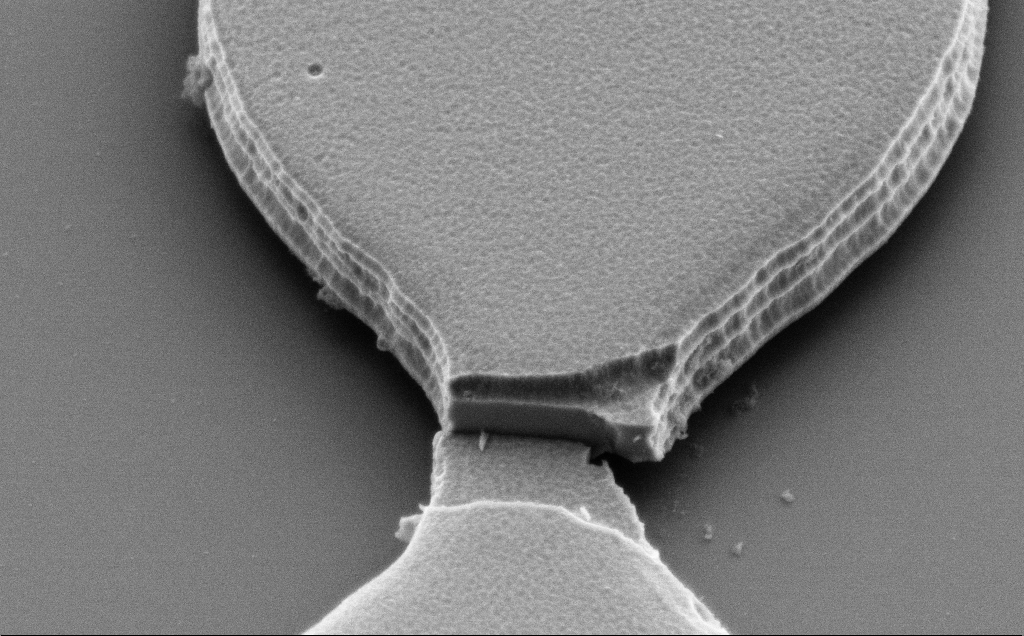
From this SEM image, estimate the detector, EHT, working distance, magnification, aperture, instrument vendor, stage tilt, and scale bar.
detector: SE2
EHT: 4 kV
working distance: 8 mm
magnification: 16.34 K X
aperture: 30 µm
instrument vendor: Zeiss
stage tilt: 45°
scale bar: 2000 nm